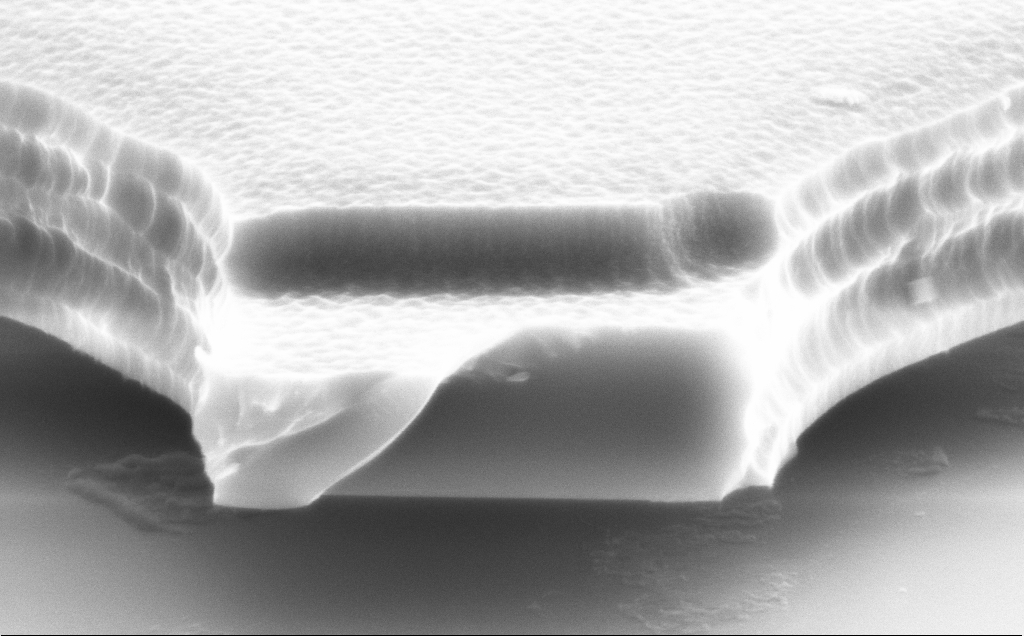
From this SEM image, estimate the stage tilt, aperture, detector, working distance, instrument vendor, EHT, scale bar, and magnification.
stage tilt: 70°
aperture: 30 µm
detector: SE2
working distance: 12 mm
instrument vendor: Zeiss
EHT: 8 kV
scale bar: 1000 nm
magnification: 42.53 K X